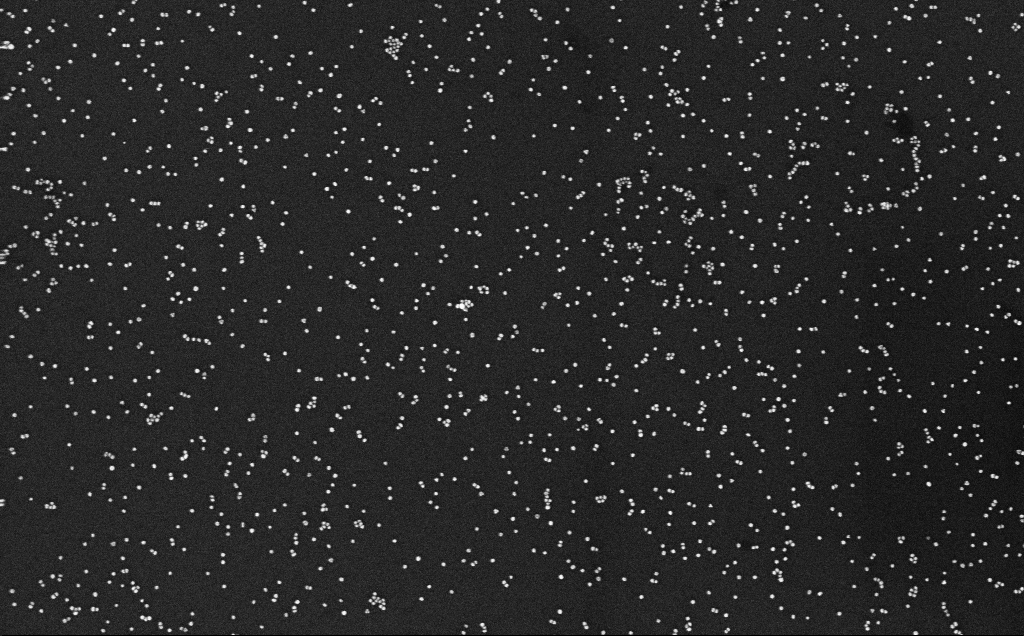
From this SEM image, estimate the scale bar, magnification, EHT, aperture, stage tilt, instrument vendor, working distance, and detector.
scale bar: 200 nm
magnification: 100 K X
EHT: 10 kV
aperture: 30 µm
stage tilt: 0°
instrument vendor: Zeiss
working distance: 3.1 mm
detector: InLens